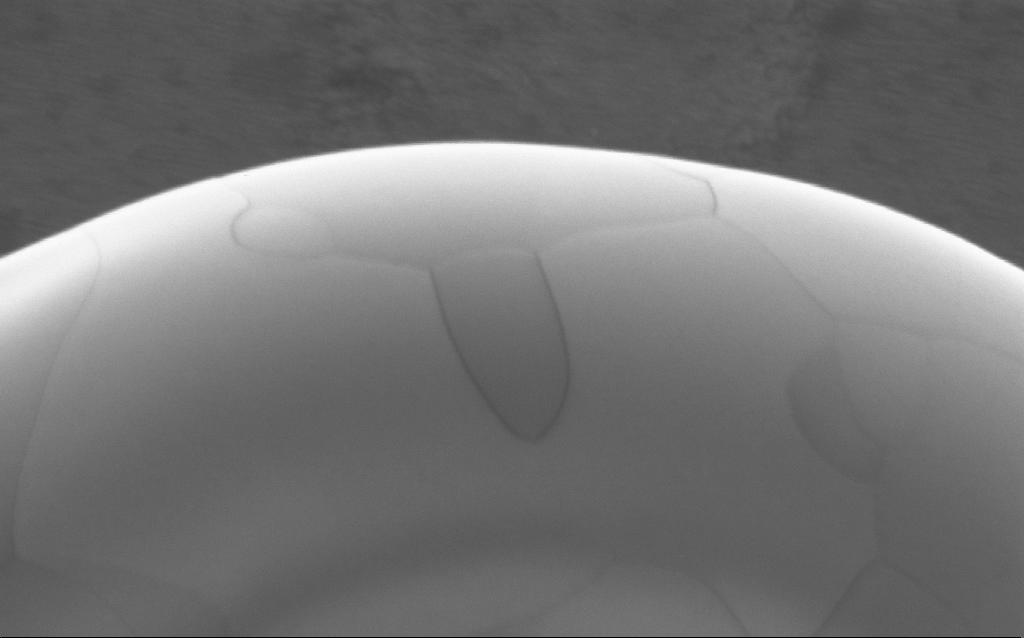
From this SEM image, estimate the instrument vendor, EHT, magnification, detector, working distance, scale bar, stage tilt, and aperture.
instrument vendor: Zeiss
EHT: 5 kV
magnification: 180.63 K X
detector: InLens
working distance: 4 mm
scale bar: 200 nm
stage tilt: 0°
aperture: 30 µm